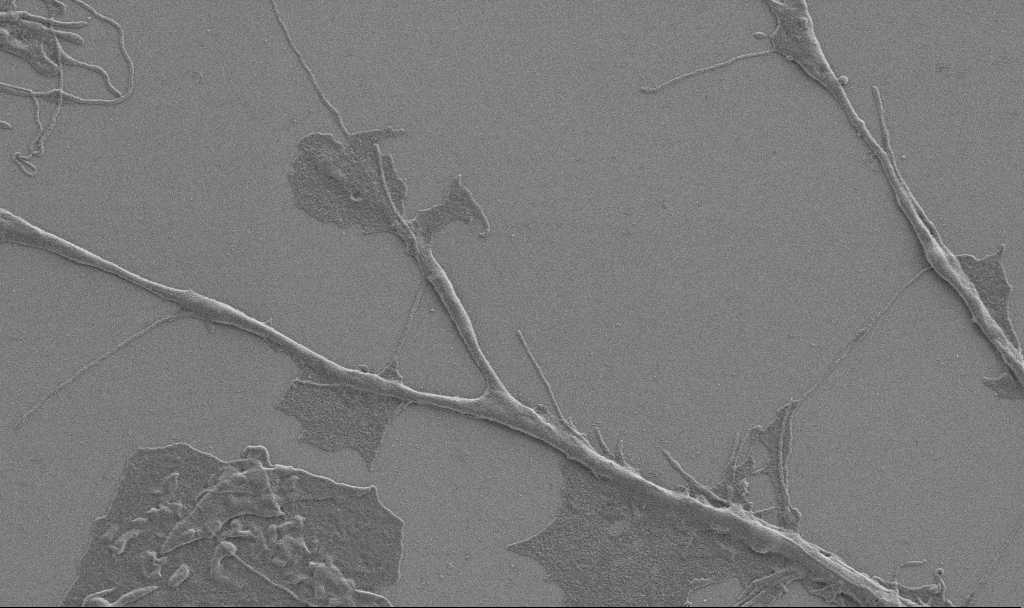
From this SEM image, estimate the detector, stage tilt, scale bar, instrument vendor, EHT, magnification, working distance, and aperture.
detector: SE2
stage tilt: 0°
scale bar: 2000 nm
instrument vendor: Zeiss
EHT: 0.9 kV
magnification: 10 K X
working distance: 6.9 mm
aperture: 30 µm